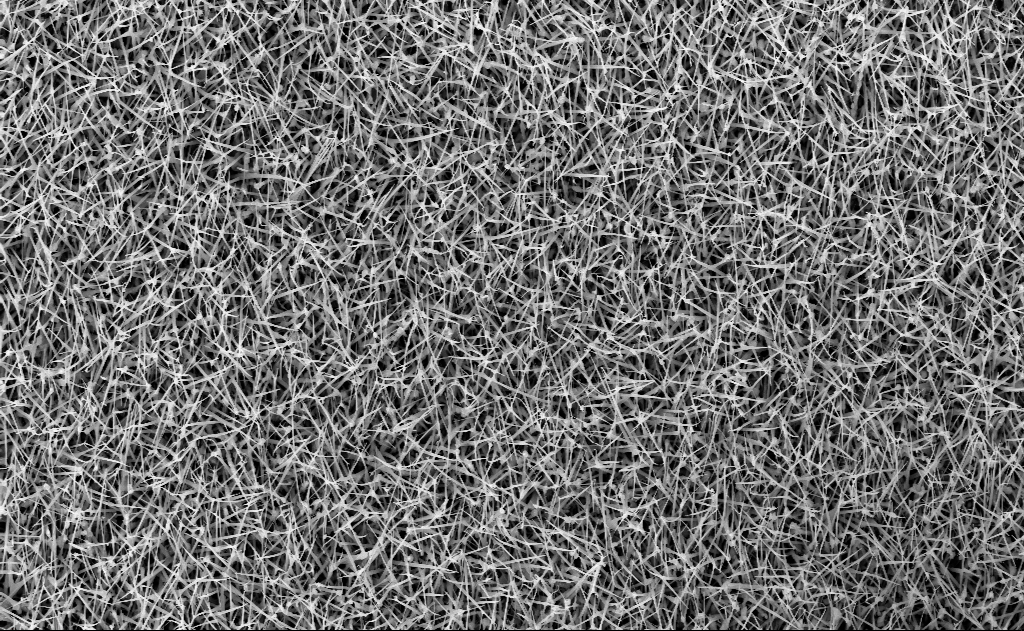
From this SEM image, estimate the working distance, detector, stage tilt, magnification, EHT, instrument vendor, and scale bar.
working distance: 10 mm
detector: InLens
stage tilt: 0°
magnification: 10 K X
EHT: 10 kV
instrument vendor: Zeiss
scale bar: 2000 nm